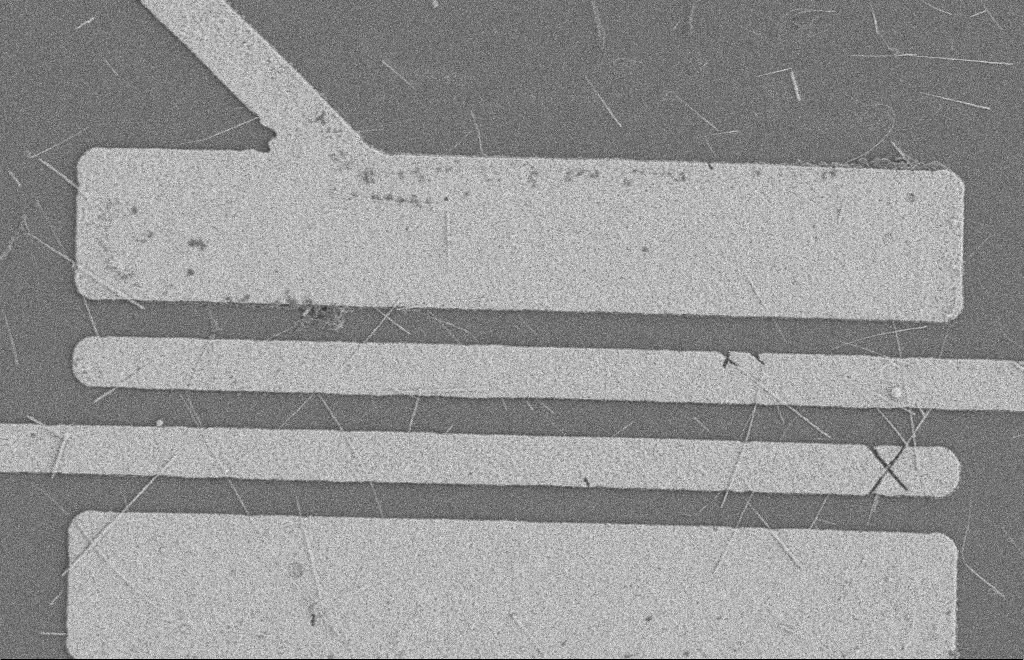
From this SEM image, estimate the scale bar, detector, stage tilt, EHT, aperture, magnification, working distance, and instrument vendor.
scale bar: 2000 nm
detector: SE2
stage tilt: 0°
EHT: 2 kV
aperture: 20 µm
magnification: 5.35 K X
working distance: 8 mm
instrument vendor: Zeiss